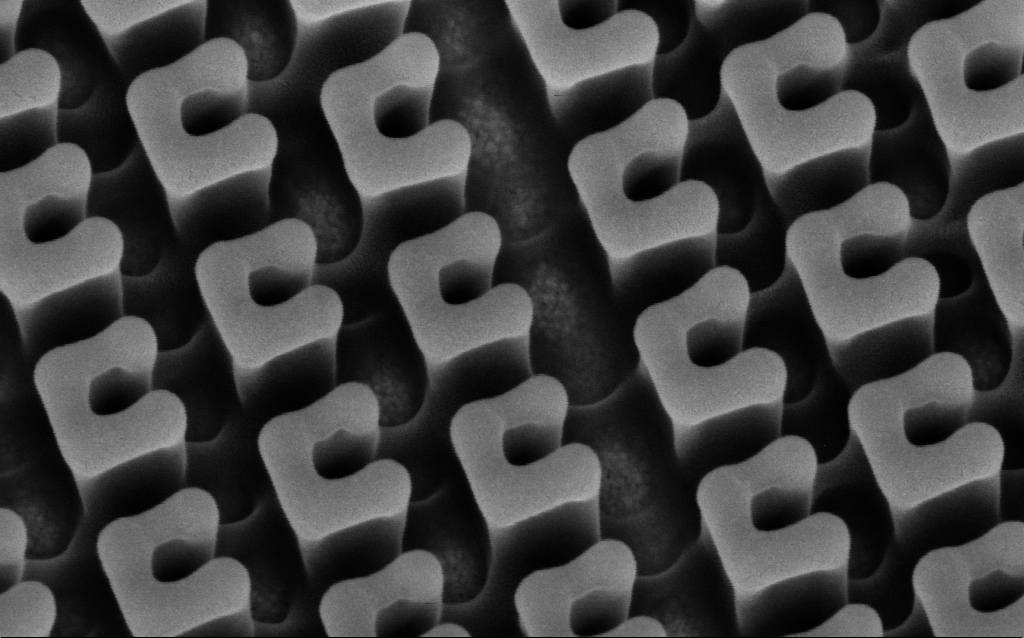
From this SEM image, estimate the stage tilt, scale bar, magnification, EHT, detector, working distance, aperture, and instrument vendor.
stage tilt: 30°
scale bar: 200 nm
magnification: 149.5 K X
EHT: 3 kV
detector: InLens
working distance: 6.3 mm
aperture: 30 µm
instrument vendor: Zeiss